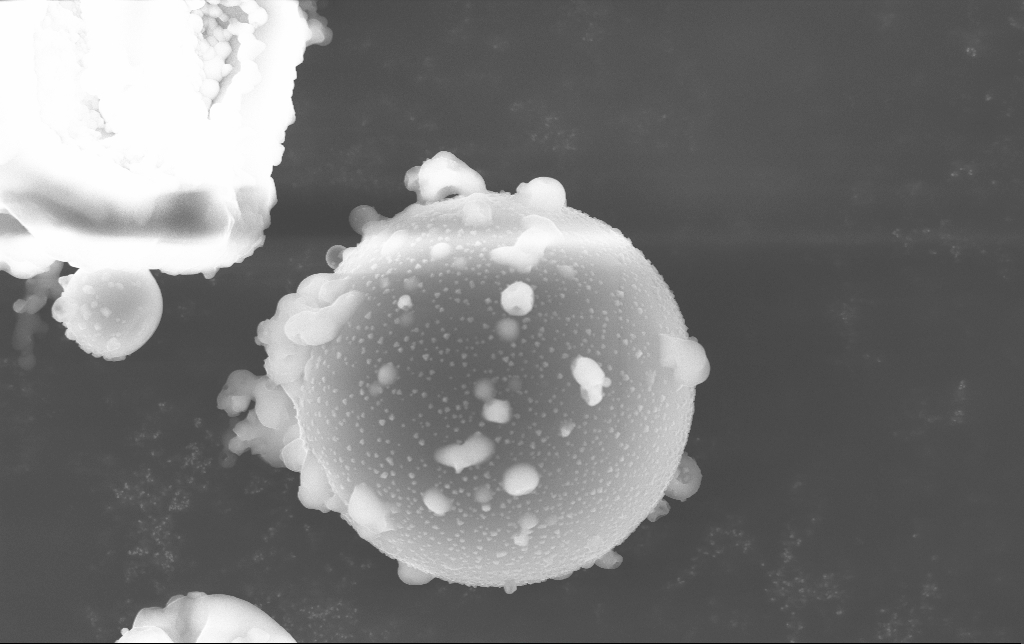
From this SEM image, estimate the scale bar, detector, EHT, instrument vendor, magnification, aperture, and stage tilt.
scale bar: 2000 nm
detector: InLens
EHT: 15 kV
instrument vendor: Zeiss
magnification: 8.57 K X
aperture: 30 µm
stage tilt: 0°